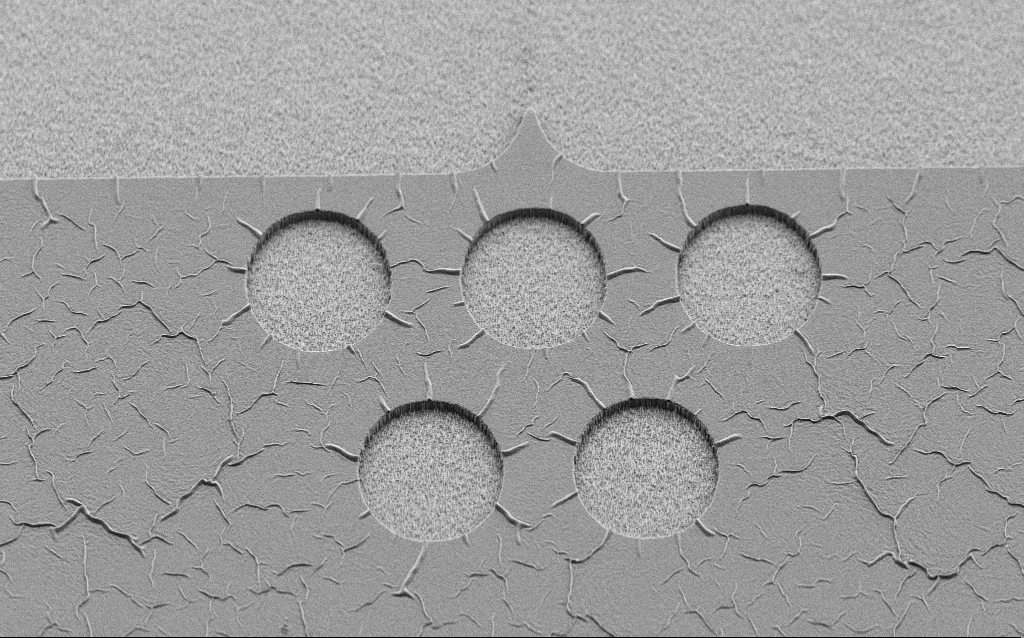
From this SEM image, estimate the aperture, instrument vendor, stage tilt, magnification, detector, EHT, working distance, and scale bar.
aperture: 30 µm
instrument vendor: Zeiss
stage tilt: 45°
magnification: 1.32 K X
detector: SE2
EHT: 3 kV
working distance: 7 mm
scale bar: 10000 nm